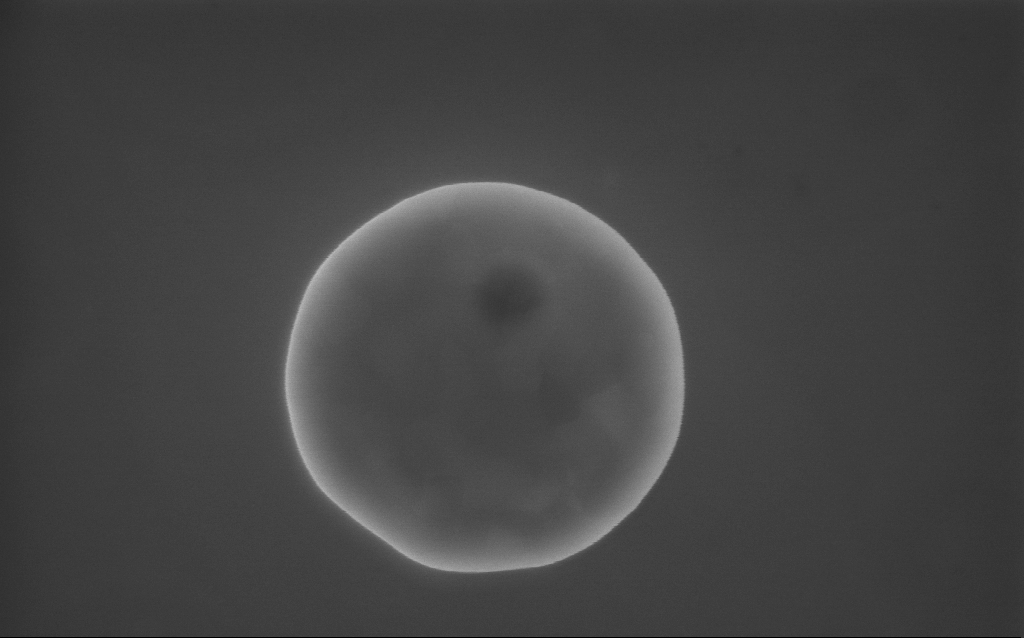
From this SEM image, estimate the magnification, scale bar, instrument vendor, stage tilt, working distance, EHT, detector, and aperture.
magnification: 115 K X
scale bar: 200 nm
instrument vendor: Zeiss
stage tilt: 0°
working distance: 3 mm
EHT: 10 kV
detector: InLens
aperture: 30 µm